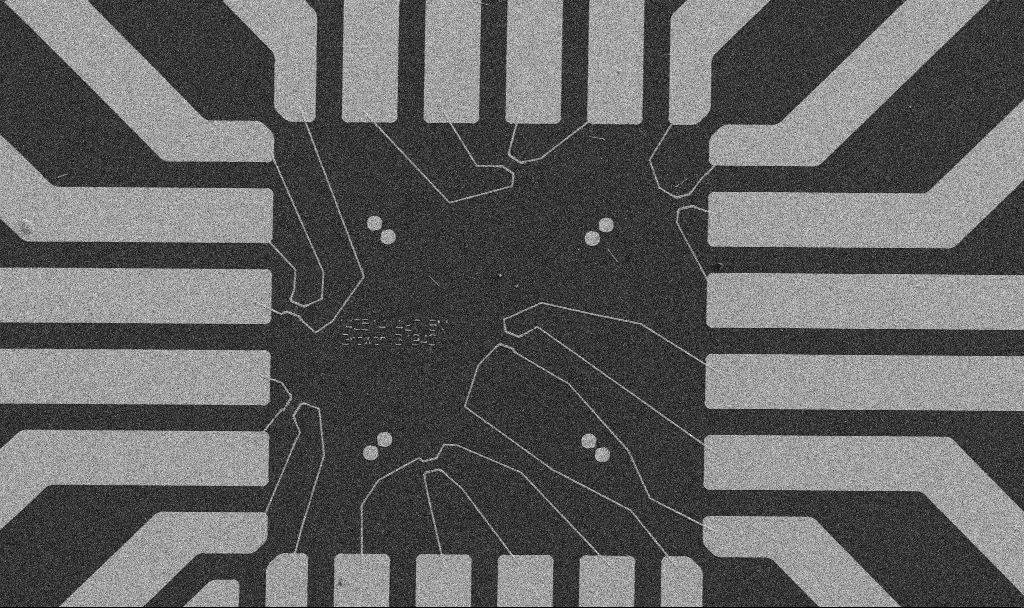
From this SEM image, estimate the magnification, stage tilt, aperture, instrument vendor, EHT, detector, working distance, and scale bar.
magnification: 1 K X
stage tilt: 0°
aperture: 30 µm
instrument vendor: Zeiss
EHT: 5 kV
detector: SE2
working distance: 10.7 mm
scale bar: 20000 nm